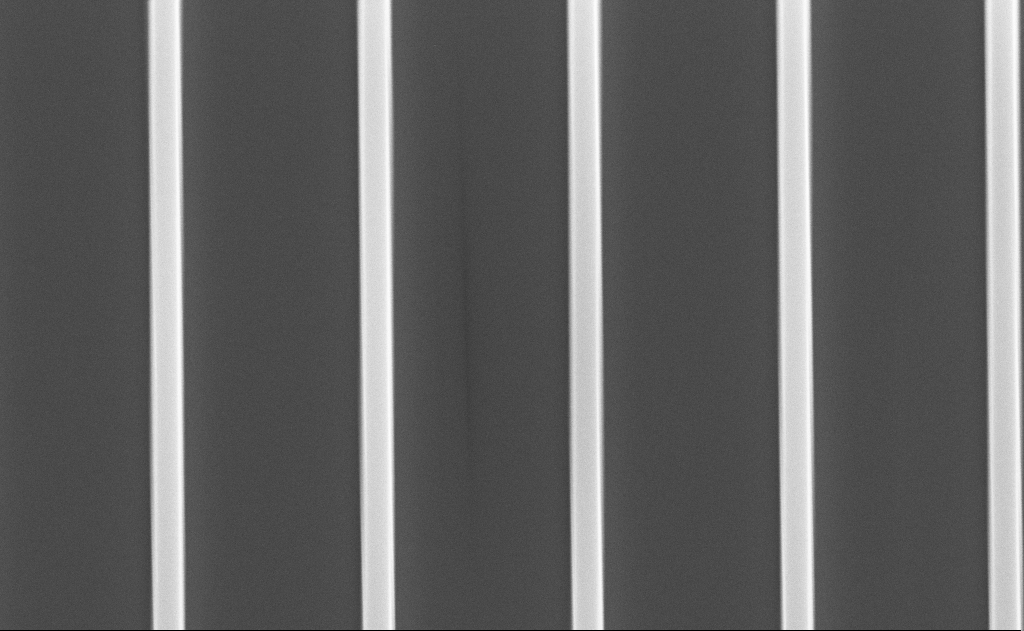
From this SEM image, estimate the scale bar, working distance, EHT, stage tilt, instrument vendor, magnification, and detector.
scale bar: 2000 nm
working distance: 14 mm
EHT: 8 kV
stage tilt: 50°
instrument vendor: Zeiss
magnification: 19.26 K X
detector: SE2